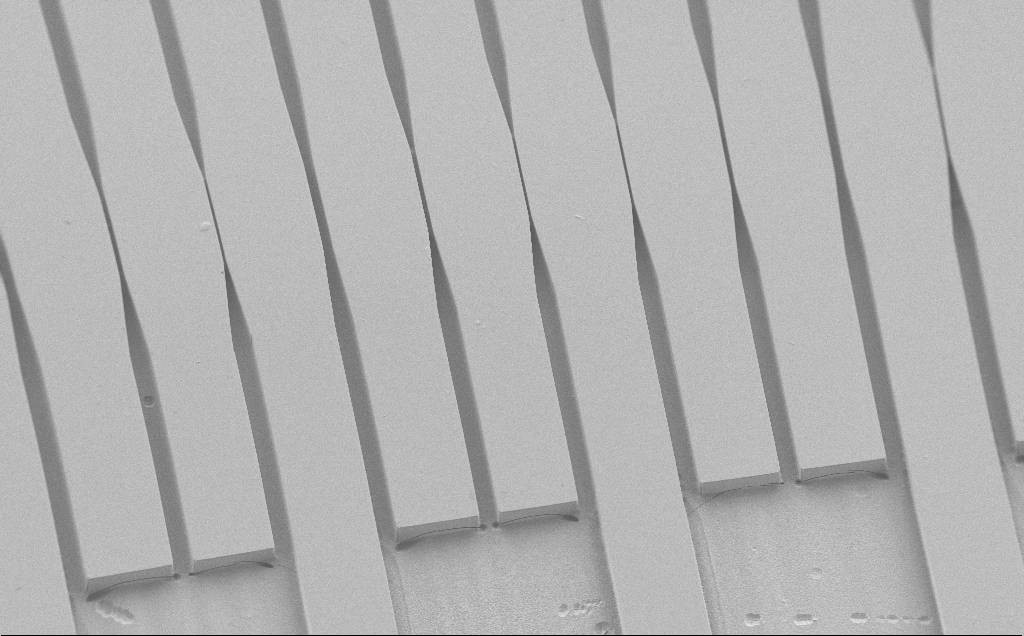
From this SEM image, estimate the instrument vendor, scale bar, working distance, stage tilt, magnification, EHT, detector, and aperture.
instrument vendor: Zeiss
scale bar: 100000 nm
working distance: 7 mm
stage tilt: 30°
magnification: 0.354 K X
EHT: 1.2 kV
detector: SE2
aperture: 30 µm